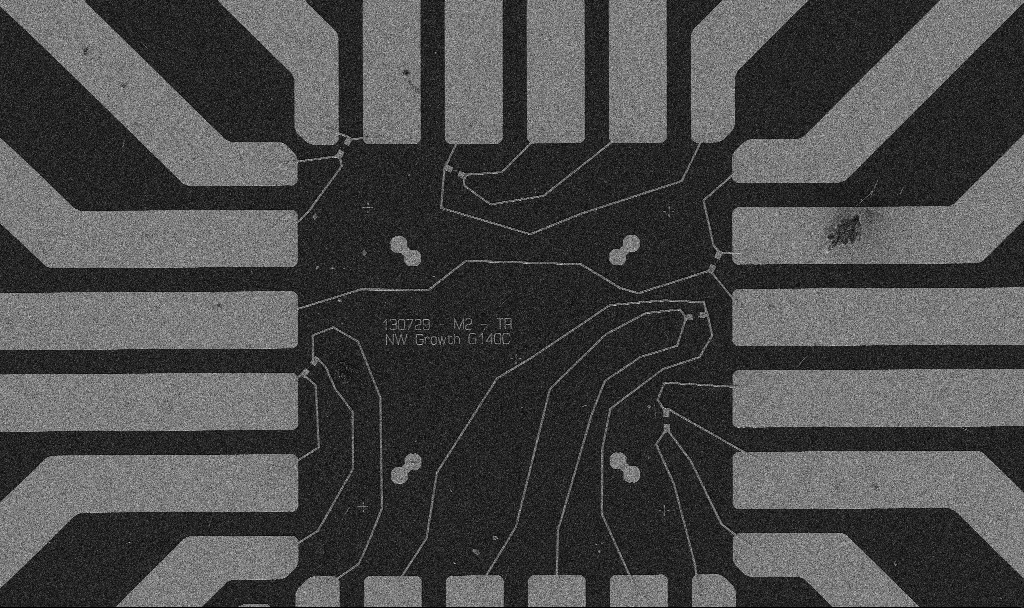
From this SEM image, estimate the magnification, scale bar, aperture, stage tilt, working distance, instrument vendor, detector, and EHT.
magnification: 1 K X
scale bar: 20000 nm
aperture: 30 µm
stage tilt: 0°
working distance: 10.7 mm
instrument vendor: Zeiss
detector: SE2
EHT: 5 kV